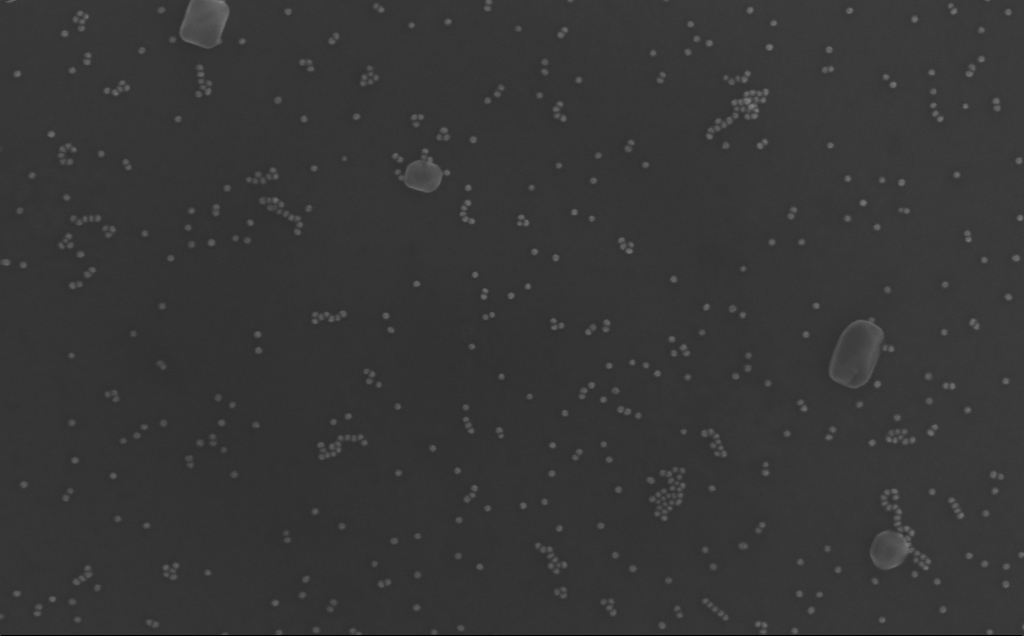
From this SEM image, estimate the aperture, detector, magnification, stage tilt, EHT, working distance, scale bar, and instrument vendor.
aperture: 30 µm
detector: InLens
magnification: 132.84 K X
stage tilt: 0°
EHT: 10 kV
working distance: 5 mm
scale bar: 200 nm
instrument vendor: Zeiss